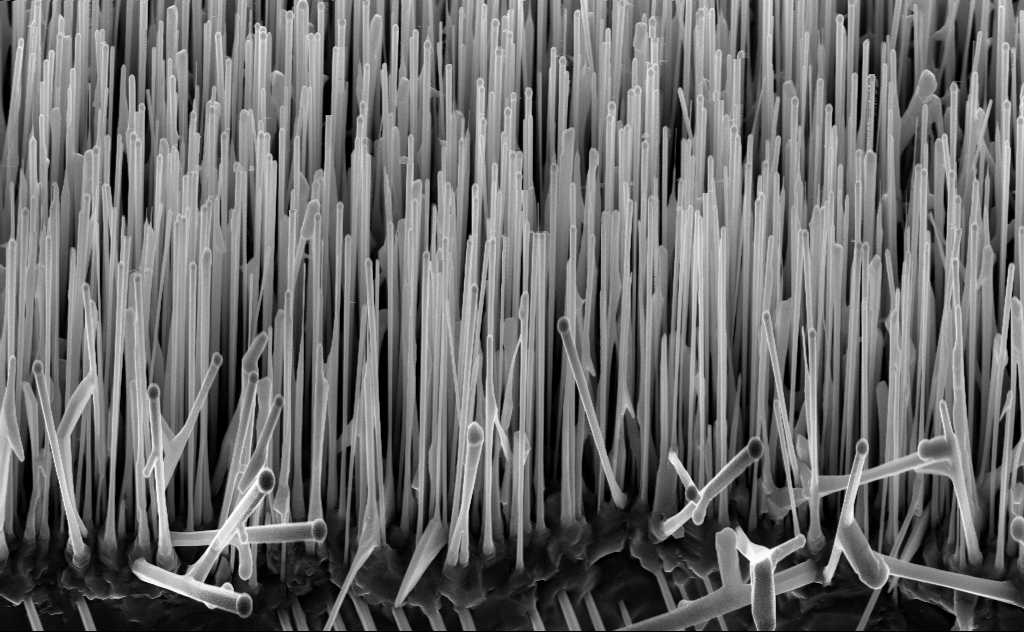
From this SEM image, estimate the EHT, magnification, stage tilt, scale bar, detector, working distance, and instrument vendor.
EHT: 10 kV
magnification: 20 K X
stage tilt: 45°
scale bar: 2000 nm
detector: InLens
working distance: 6 mm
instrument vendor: Zeiss